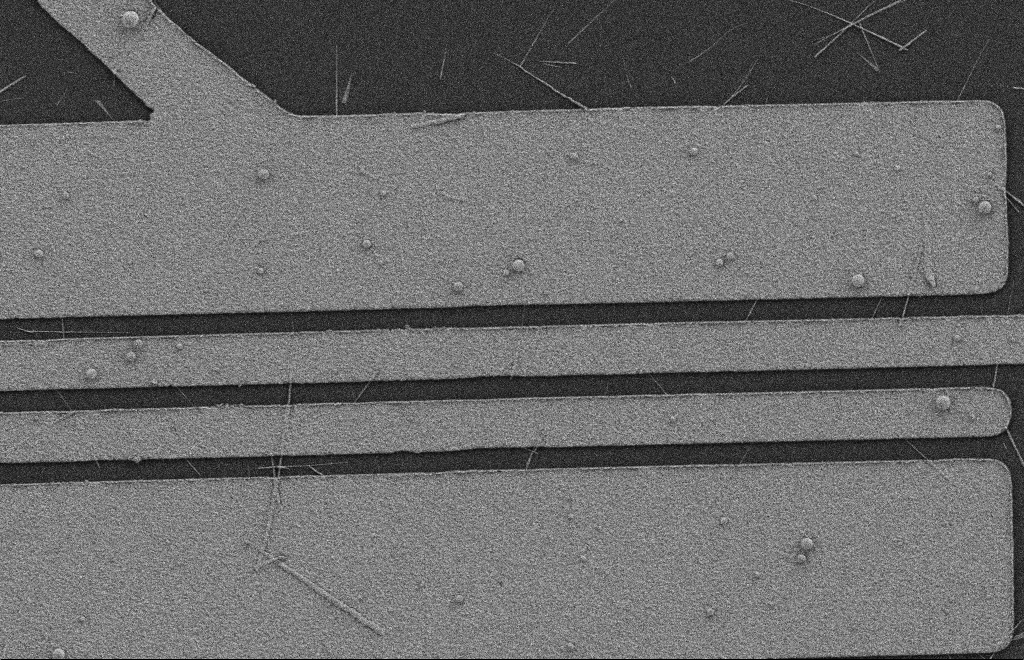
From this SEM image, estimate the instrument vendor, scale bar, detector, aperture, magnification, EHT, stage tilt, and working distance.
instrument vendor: Zeiss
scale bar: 2000 nm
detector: SE2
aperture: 20 µm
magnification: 6.61 K X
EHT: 2 kV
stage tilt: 0°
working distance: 9 mm